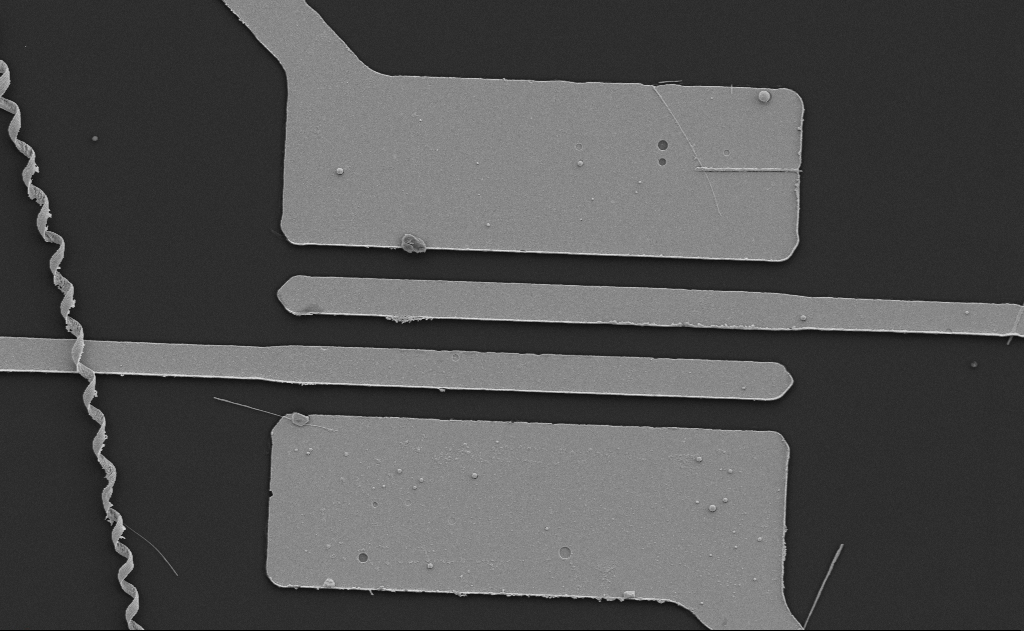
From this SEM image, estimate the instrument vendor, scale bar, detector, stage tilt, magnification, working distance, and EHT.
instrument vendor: Zeiss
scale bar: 10000 nm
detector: SE2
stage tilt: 0°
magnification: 6.34 K X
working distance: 13 mm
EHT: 5 kV